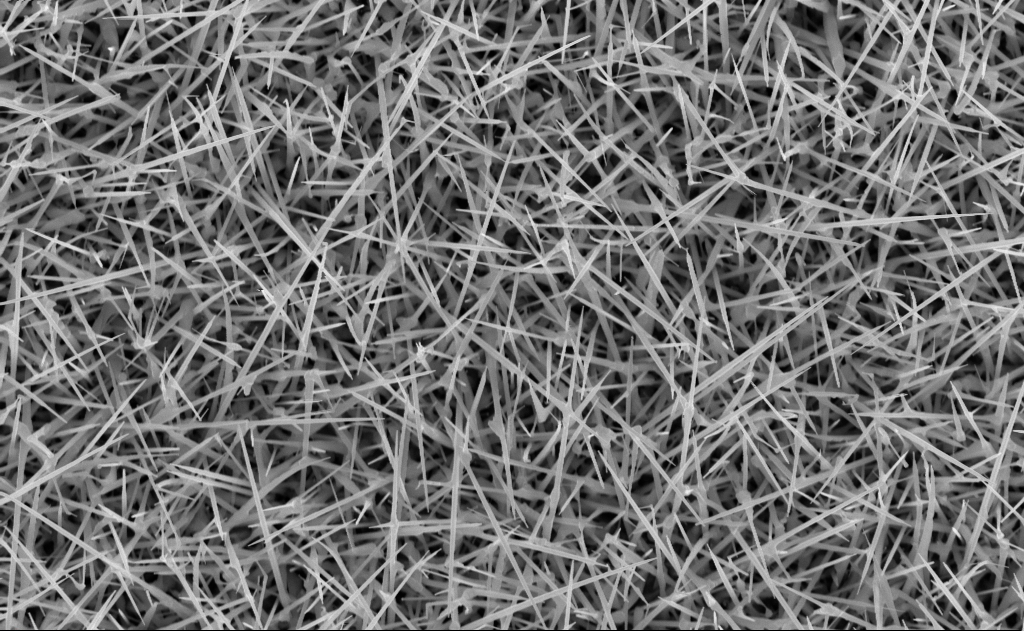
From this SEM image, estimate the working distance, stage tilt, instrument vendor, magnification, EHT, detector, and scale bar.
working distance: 10 mm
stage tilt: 0°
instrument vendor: Zeiss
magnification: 20 K X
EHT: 10 kV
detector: InLens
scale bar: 2000 nm